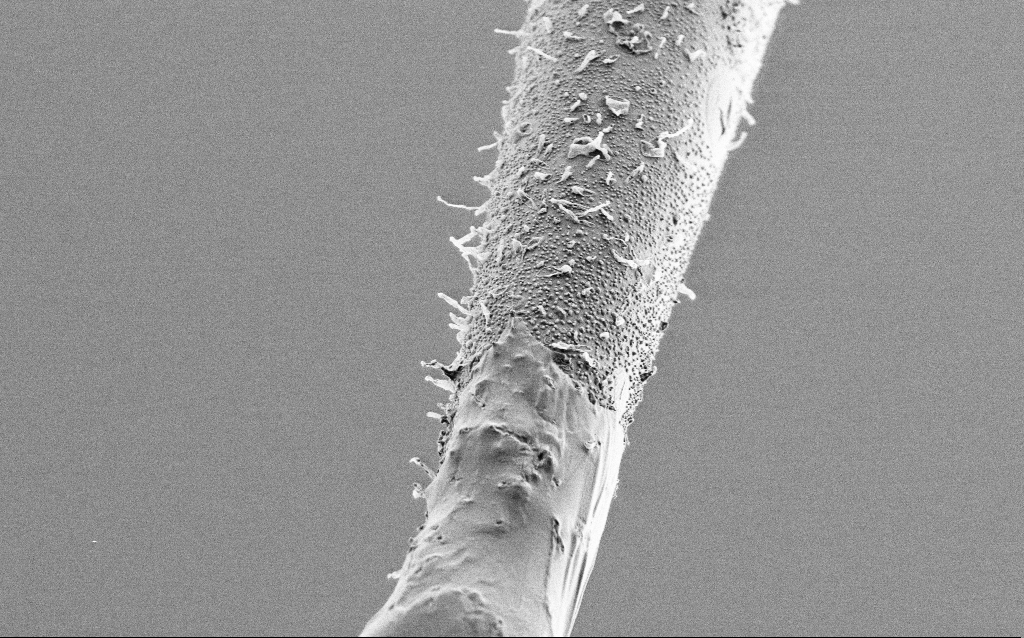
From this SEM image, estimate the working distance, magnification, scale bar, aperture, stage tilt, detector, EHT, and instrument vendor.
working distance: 6.6 mm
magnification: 10 K X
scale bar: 2000 nm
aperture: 30 µm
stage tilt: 45°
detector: SE2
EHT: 1 kV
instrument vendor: Zeiss